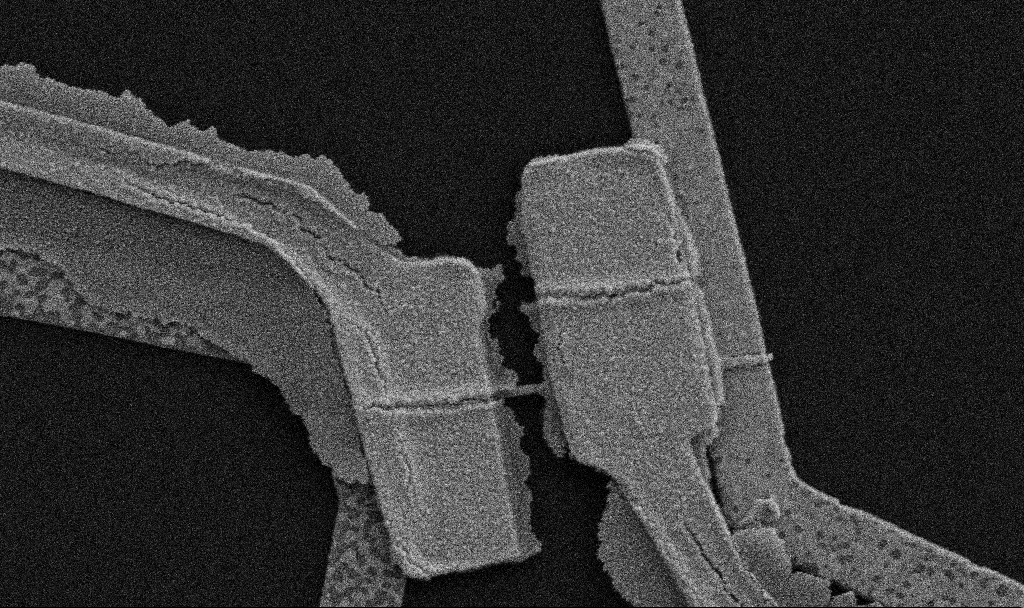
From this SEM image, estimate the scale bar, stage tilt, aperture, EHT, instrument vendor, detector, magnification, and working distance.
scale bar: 1000 nm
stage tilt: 0°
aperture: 30 µm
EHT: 5 kV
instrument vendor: Zeiss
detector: SE2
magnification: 30 K X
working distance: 10.7 mm